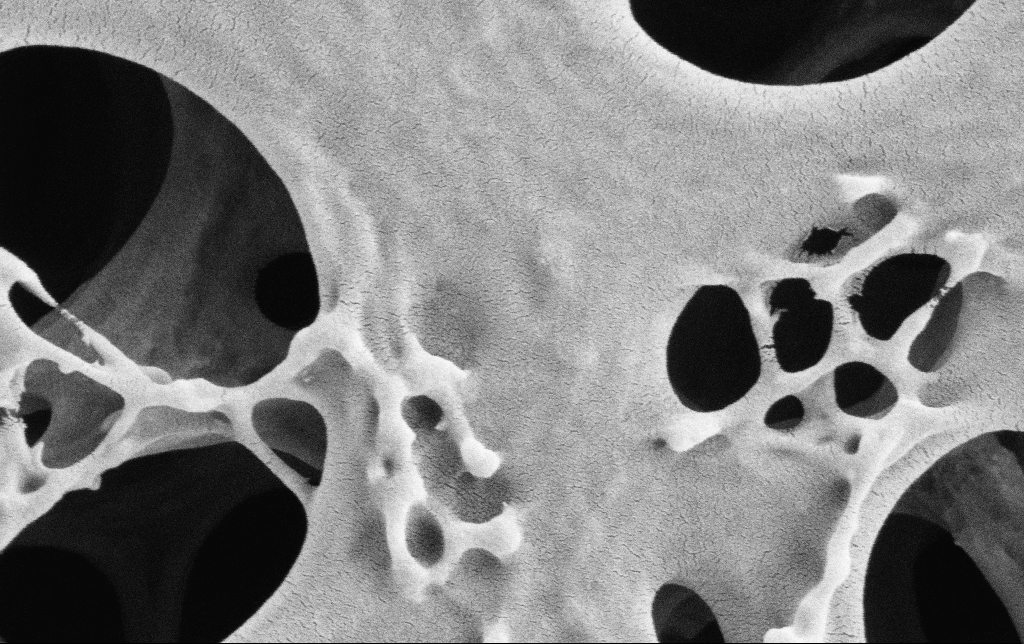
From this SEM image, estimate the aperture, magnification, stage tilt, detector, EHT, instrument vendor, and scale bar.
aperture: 30 µm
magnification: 100 K X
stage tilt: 0°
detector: SE2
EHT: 2 kV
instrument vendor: Zeiss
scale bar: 200 nm